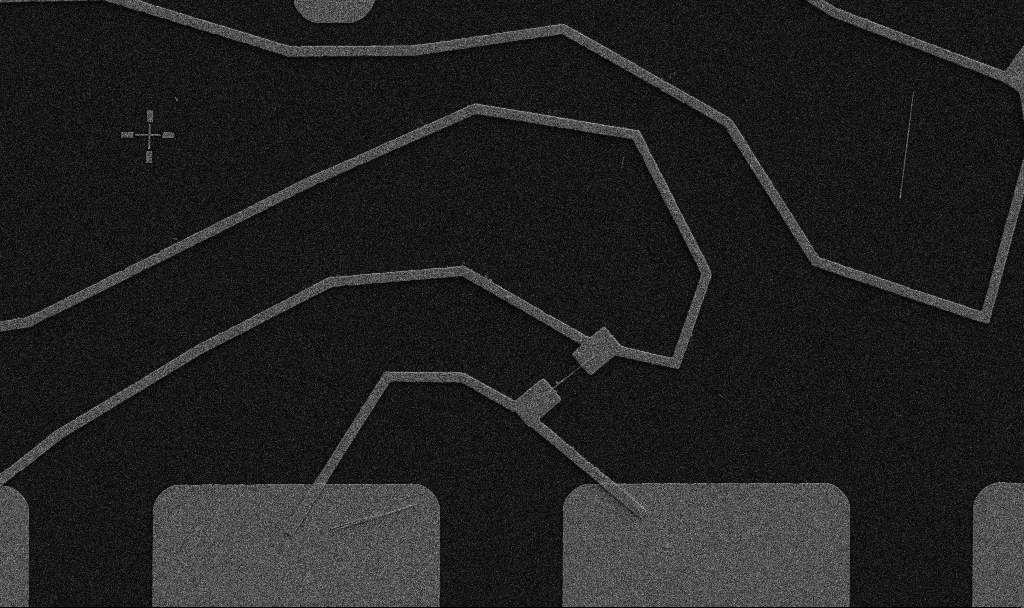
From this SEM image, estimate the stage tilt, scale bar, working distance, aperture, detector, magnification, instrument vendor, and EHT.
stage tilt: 0°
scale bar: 10000 nm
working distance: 10.7 mm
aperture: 30 µm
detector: SE2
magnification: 5 K X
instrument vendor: Zeiss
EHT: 5 kV